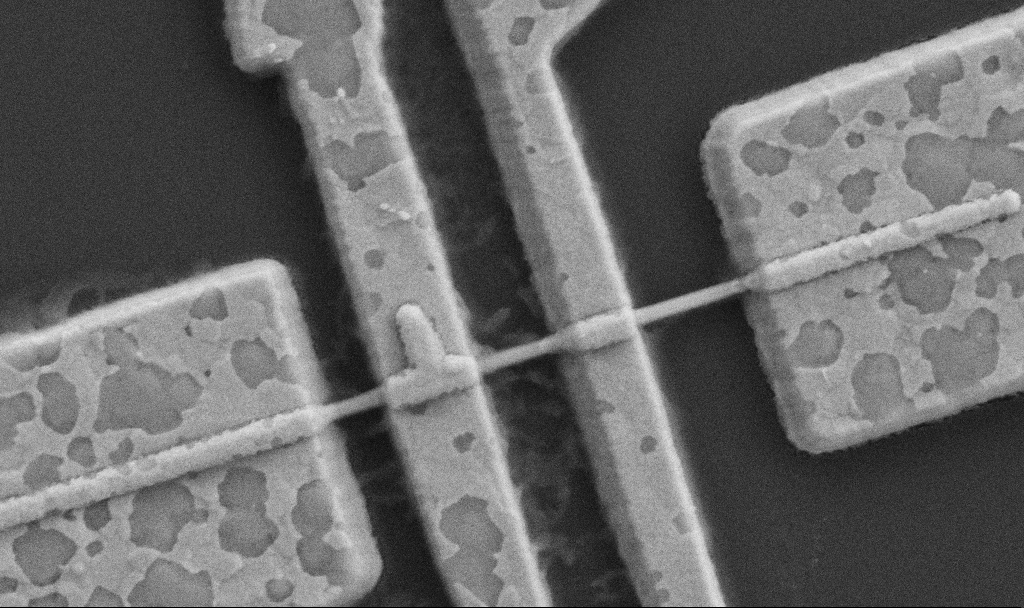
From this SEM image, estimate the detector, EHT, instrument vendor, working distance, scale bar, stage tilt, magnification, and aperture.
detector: SE2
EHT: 5 kV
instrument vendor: Zeiss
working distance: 8.7 mm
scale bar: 1000 nm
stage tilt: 0°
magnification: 60 K X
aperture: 30 µm